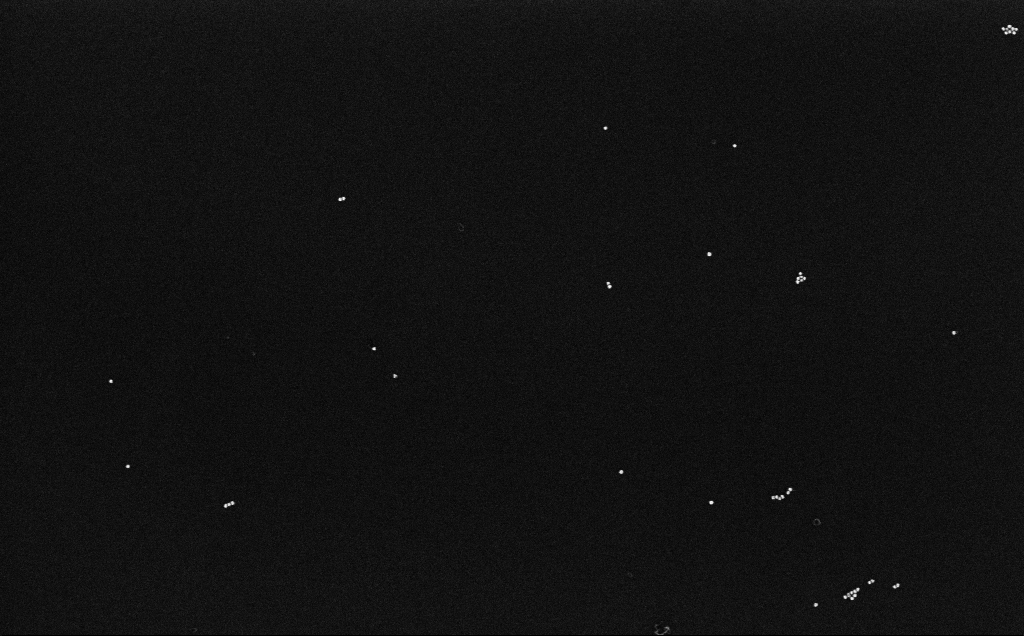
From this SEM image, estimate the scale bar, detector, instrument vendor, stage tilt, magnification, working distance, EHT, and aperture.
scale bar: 200 nm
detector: InLens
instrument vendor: Zeiss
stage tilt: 0°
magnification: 100 K X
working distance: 6.6 mm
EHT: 10 kV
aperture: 30 µm